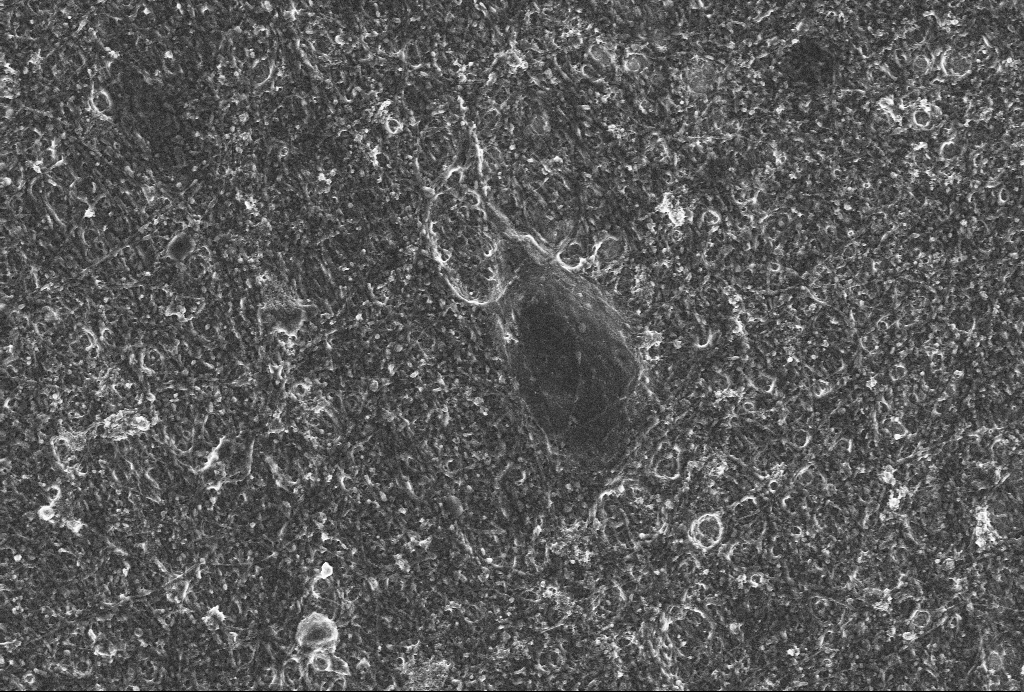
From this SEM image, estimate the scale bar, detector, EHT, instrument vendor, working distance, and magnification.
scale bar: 10000 nm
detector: InLens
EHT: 4 kV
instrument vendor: Zeiss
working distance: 6 mm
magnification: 5 K X